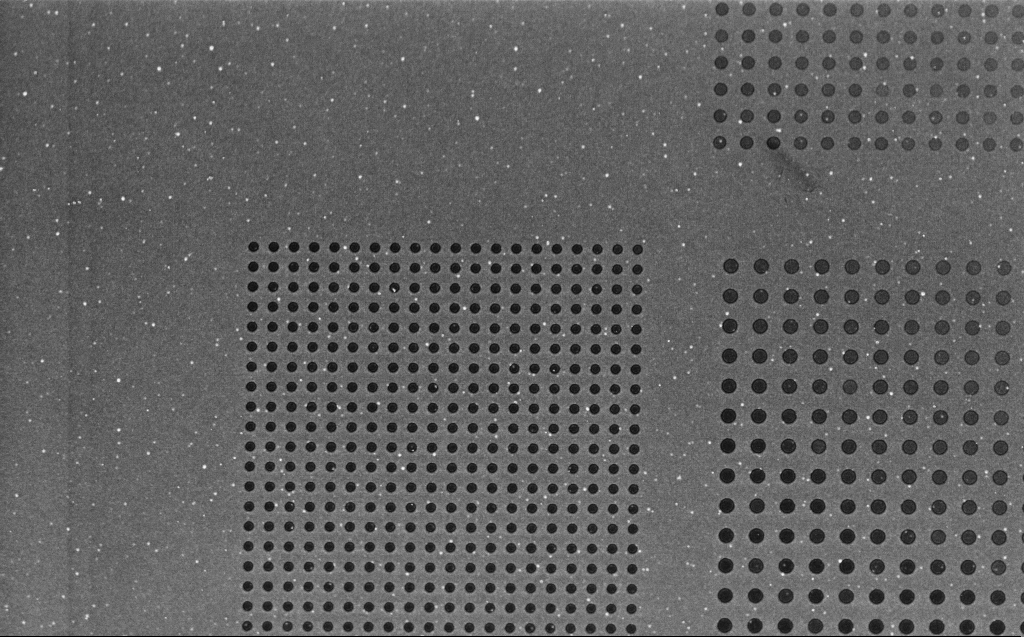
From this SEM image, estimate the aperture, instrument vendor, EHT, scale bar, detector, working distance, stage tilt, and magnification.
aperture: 30 µm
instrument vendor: Zeiss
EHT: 1 kV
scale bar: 10000 nm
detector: InLens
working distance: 4 mm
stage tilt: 30°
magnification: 6.26 K X